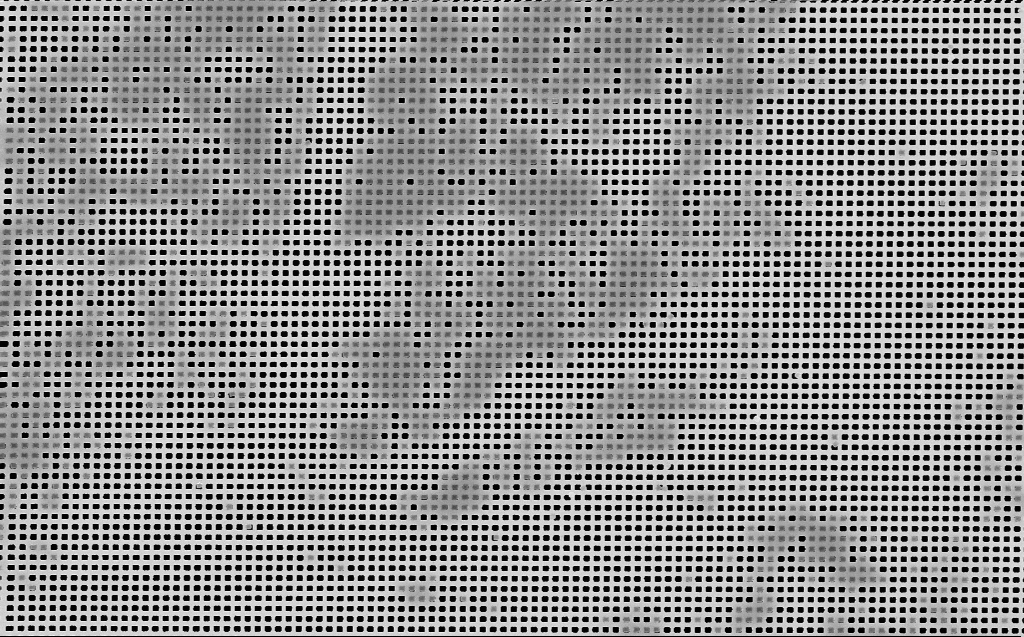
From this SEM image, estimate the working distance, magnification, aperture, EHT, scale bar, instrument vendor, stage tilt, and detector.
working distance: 7 mm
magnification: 7.59 K X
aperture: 30 µm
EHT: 10 kV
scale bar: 2000 nm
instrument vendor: Zeiss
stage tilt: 0°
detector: InLens